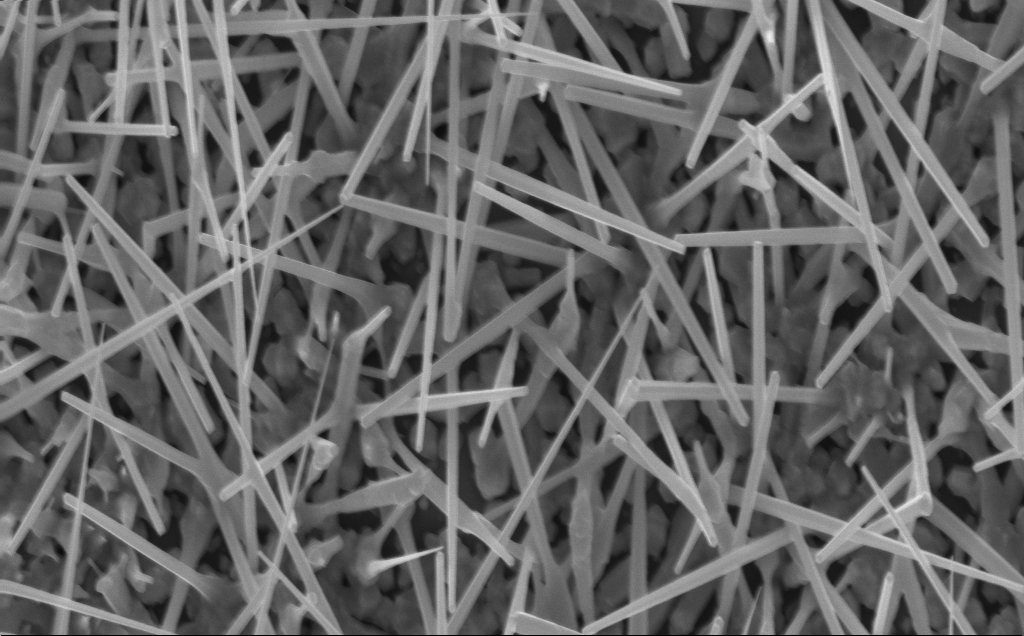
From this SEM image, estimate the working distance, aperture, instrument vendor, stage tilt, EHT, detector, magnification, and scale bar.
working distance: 4 mm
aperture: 30 µm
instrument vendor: Zeiss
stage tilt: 0°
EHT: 10 kV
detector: InLens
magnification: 20 K X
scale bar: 2000 nm